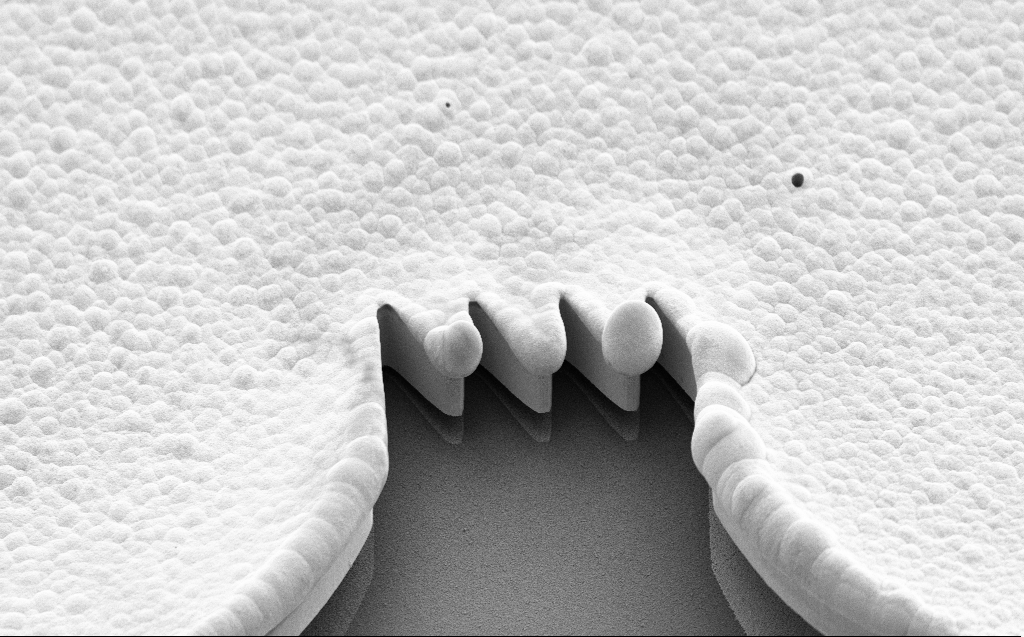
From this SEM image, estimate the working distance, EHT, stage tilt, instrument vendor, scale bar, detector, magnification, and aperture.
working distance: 9 mm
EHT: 5 kV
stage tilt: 45°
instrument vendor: Zeiss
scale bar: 10000 nm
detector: SE2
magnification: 2.84 K X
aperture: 30 µm